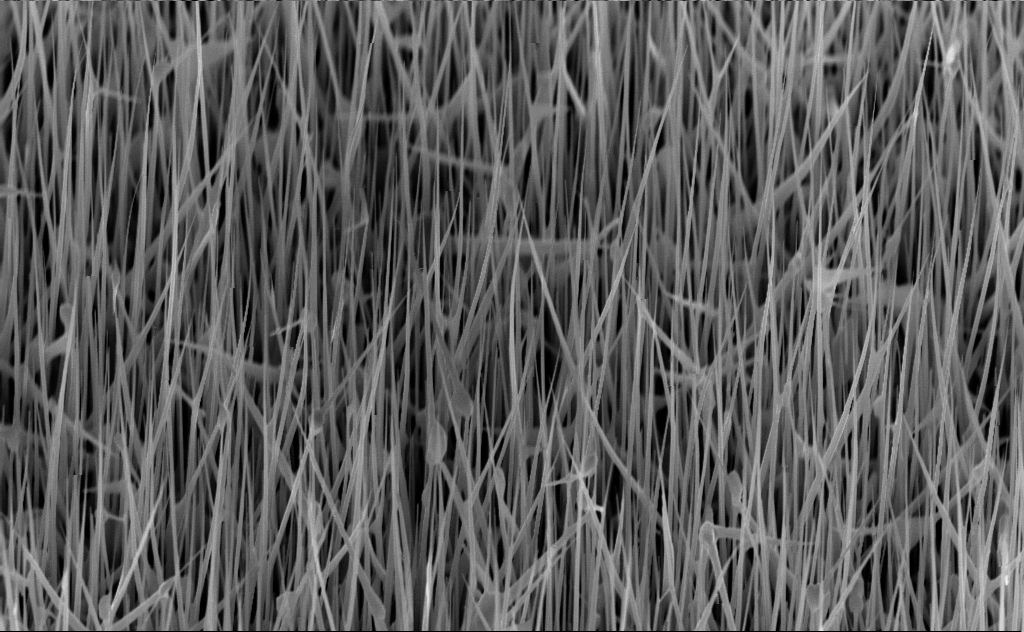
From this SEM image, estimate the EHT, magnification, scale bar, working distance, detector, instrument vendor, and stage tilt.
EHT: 10 kV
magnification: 20 K X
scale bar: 2000 nm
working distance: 6 mm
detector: InLens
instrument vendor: Zeiss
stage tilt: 45°